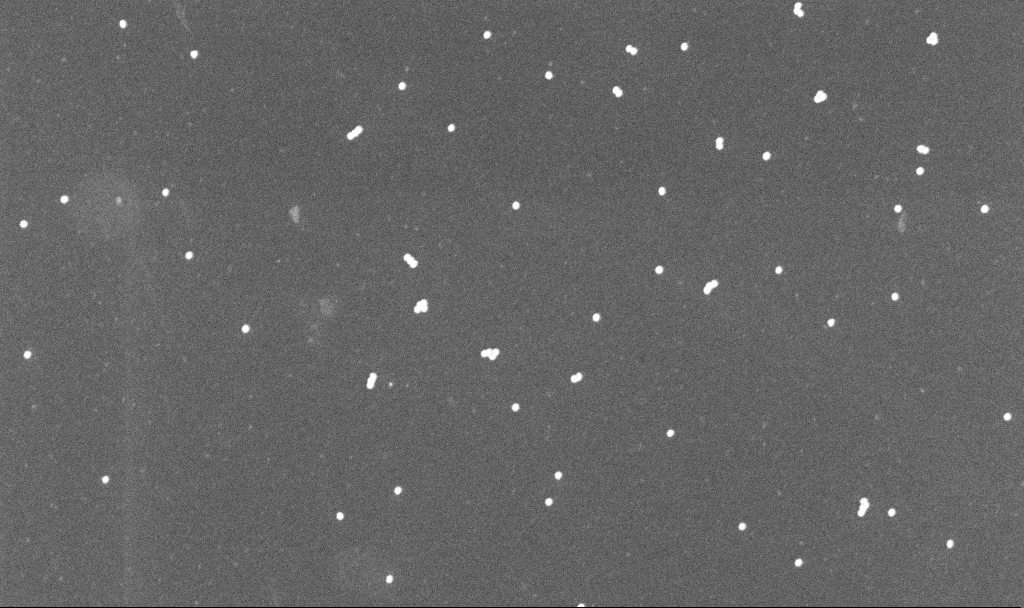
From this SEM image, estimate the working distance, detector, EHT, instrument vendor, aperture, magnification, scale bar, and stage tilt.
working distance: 3.3 mm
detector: InLens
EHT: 10 kV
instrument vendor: Zeiss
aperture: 30 µm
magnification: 100 K X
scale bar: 200 nm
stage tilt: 0°